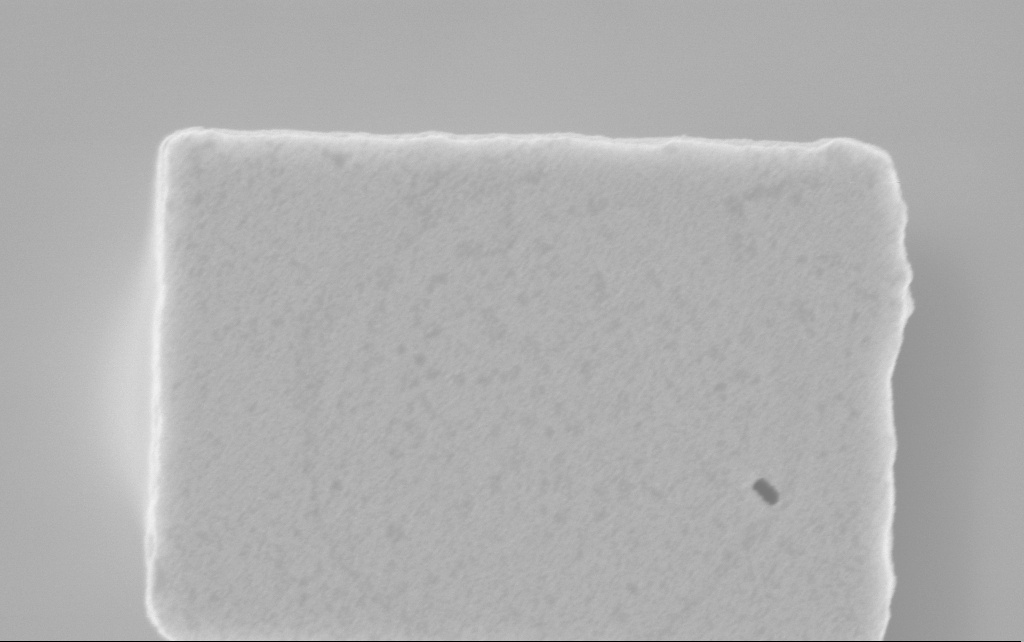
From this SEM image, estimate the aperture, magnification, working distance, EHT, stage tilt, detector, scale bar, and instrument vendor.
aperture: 30 µm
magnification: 89.83 K X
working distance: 2.5 mm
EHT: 3 kV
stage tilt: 0°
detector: InLens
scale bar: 200 nm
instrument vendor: Zeiss